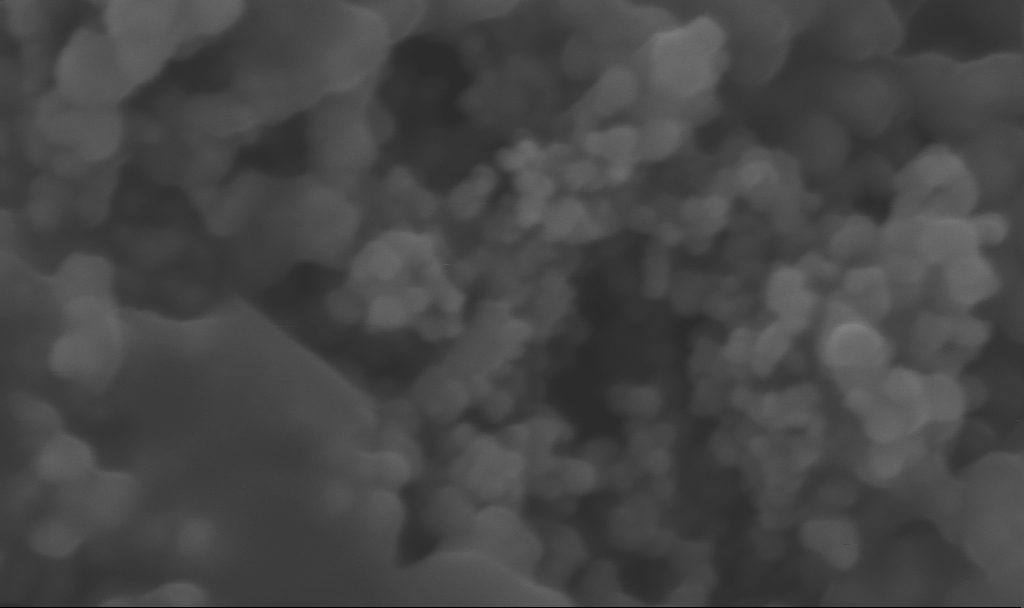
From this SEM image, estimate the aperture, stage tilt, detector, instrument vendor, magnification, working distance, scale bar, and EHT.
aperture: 30 µm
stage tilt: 0°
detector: InLens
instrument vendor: Zeiss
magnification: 423.45 K X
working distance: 2.5 mm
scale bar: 100 nm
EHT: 3 kV